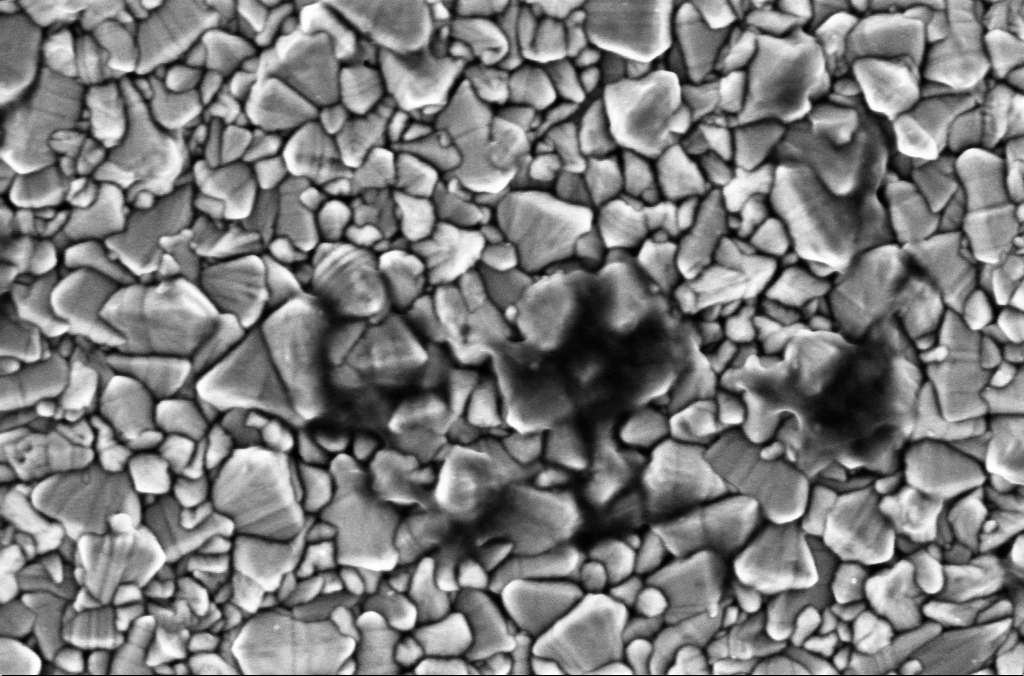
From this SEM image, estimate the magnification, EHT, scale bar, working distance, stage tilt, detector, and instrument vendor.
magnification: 100 K X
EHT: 2 kV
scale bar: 200 nm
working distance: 1.9 mm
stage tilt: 0°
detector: InLens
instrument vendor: Zeiss